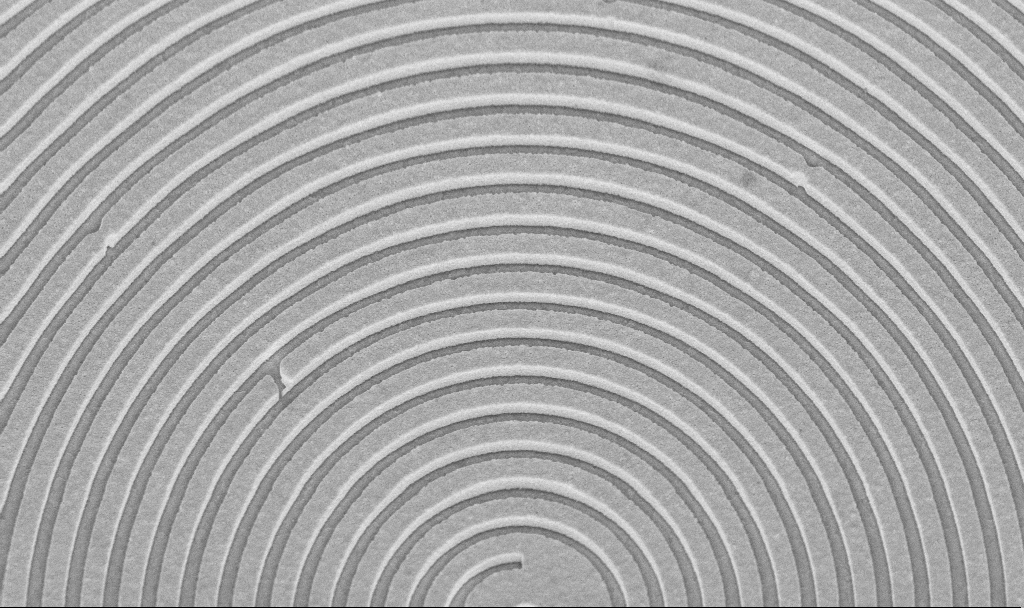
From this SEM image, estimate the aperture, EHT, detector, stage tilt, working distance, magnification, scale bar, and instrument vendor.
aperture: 30 µm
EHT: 5 kV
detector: InLens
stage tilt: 45°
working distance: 8.4 mm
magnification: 21.77 K X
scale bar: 2000 nm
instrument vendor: Zeiss